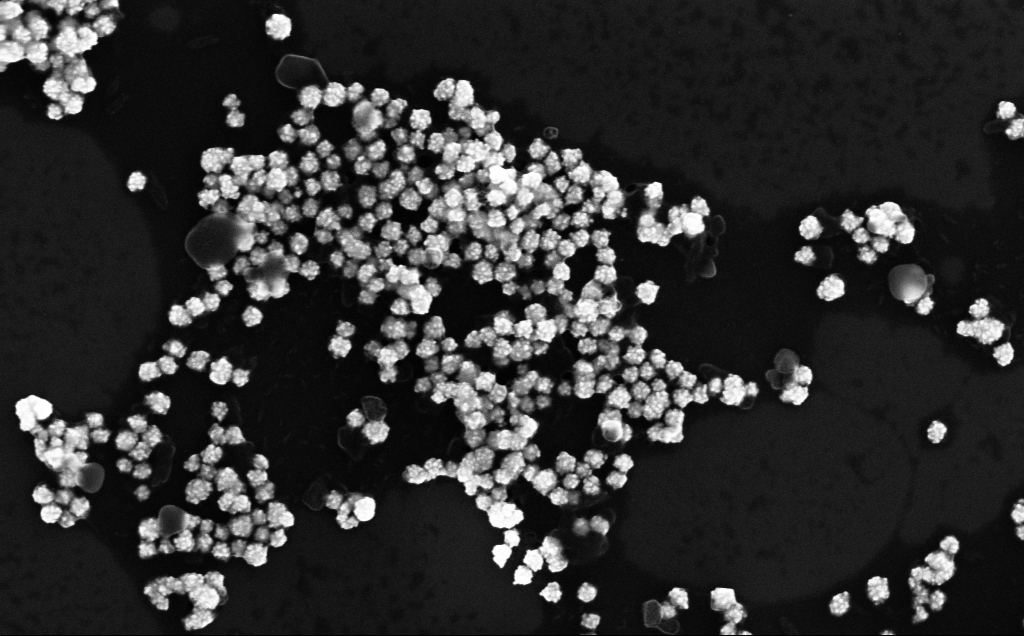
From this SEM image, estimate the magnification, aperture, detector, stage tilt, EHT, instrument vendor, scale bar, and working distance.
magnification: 110.82 K X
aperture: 30 µm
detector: InLens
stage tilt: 0°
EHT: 10 kV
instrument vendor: Zeiss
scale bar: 200 nm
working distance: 4 mm